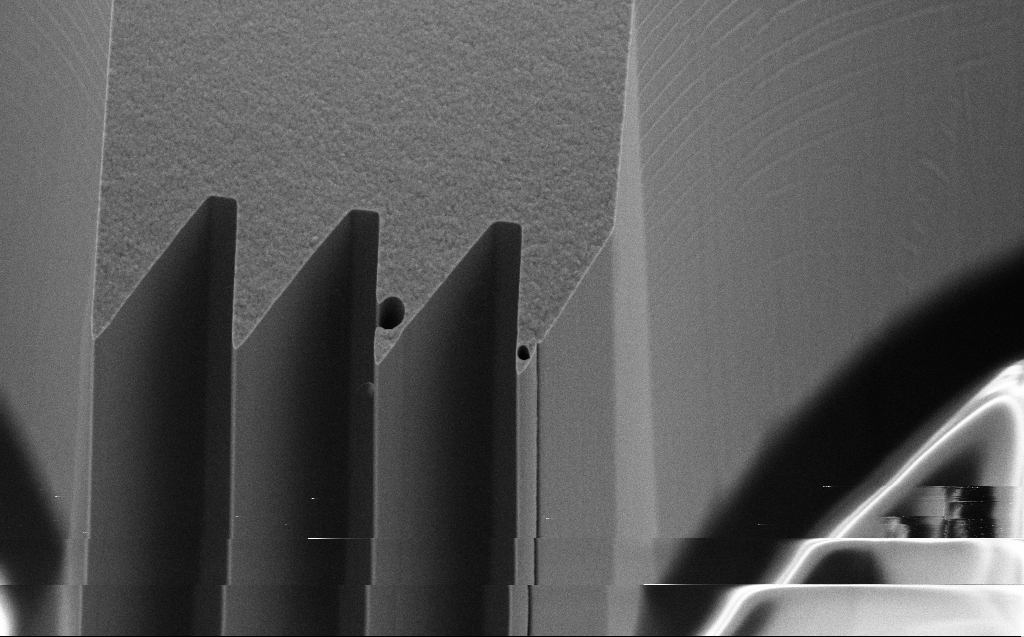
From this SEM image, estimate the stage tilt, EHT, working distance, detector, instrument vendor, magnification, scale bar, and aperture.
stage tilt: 45°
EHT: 10 kV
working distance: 7 mm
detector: SE2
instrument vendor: Zeiss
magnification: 4.59 K X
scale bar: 10000 nm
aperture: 30 µm